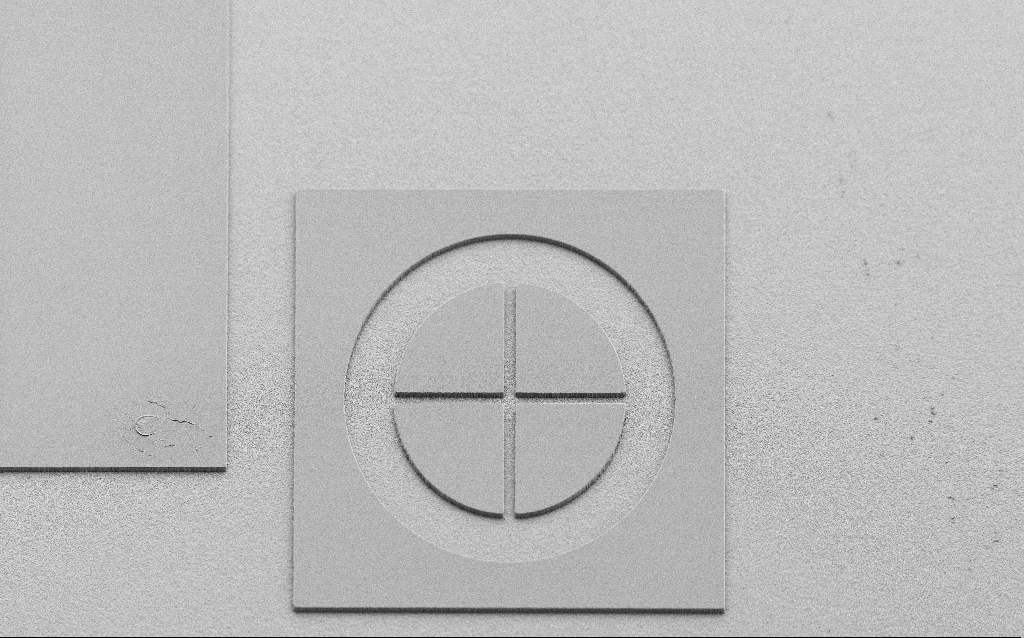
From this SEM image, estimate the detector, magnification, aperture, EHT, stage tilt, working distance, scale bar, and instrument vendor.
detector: SE2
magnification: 0.347 K X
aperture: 30 µm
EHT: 3 kV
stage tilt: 45°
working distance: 7 mm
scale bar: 100000 nm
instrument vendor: Zeiss